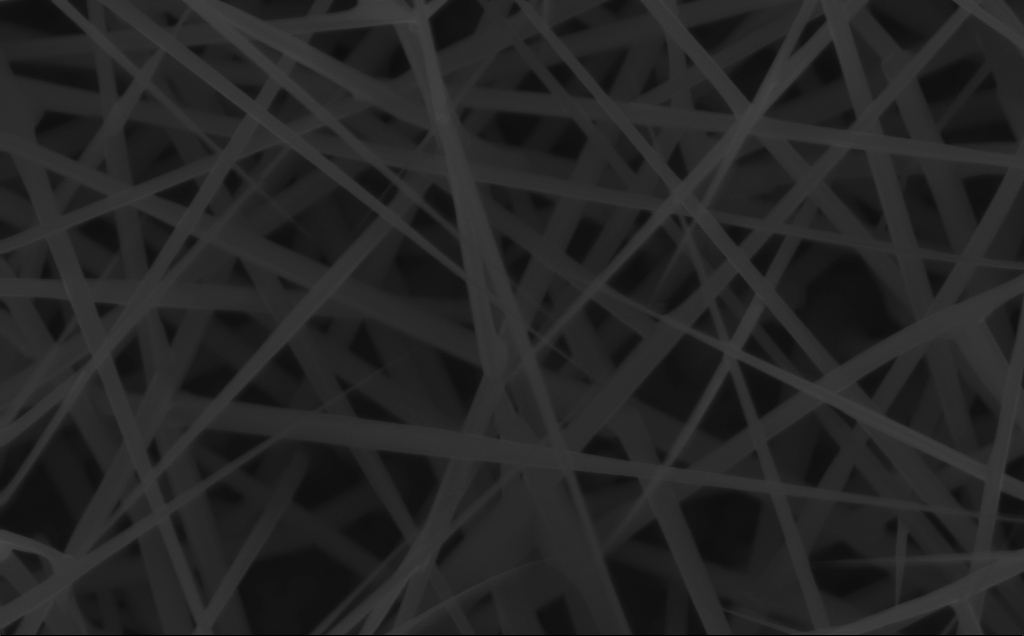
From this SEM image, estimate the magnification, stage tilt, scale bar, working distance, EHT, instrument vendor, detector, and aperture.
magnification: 80 K X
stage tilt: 0°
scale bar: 200 nm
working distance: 4 mm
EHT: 10 kV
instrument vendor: Zeiss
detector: InLens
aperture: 30 µm